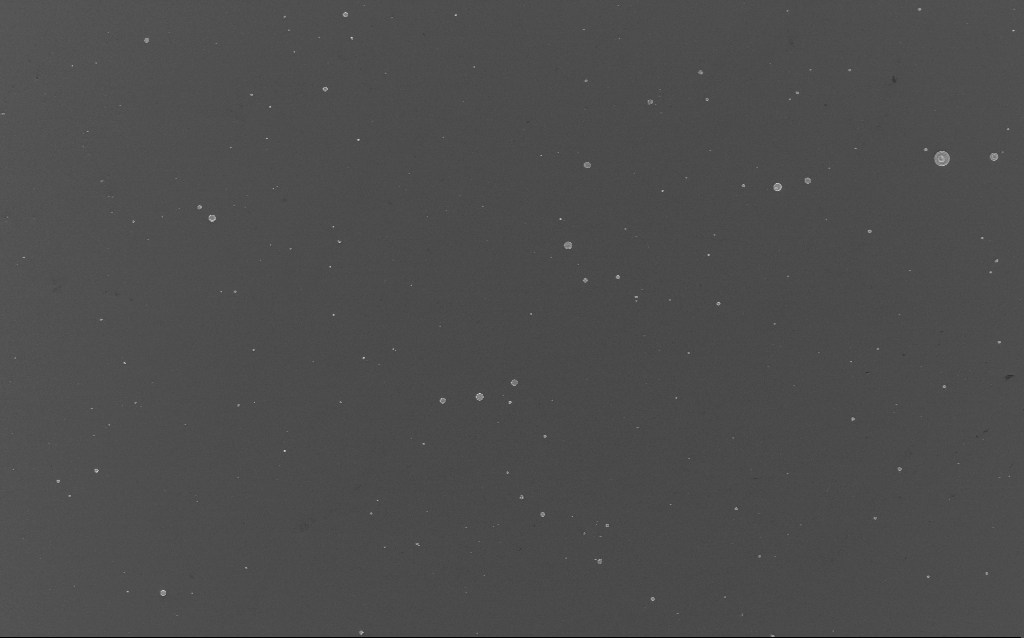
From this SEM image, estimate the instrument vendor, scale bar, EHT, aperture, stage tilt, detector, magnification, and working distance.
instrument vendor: Zeiss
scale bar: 20000 nm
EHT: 3 kV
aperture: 30 µm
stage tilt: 0°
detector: InLens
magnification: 1.18 K X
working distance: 3 mm